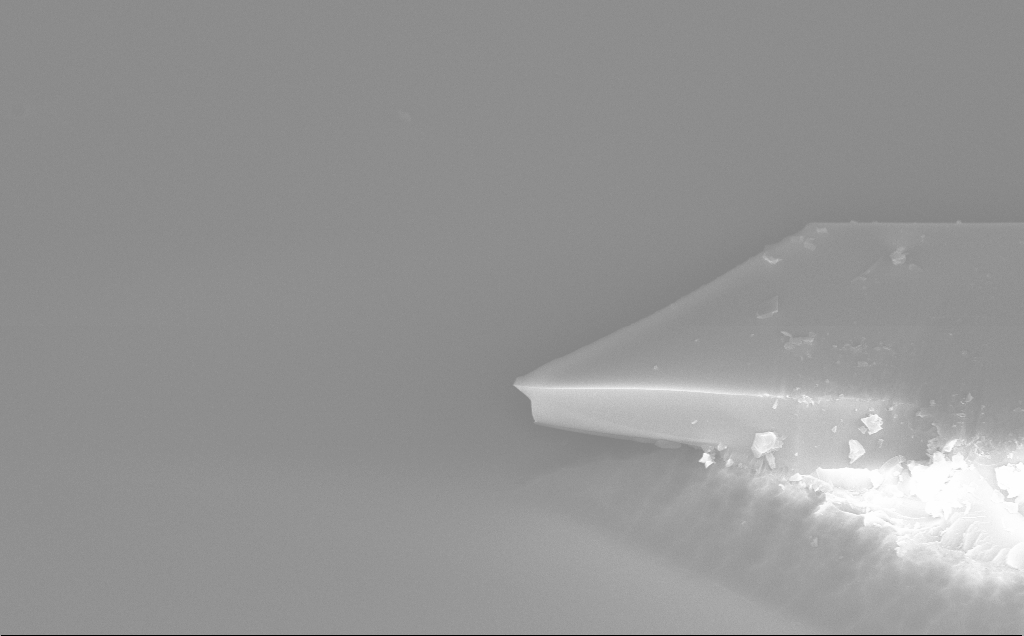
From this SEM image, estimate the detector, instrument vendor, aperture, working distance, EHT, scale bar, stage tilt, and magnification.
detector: InLens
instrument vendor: Zeiss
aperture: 30 µm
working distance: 10 mm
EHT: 10 kV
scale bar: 2000 nm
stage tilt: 50°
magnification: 10.58 K X